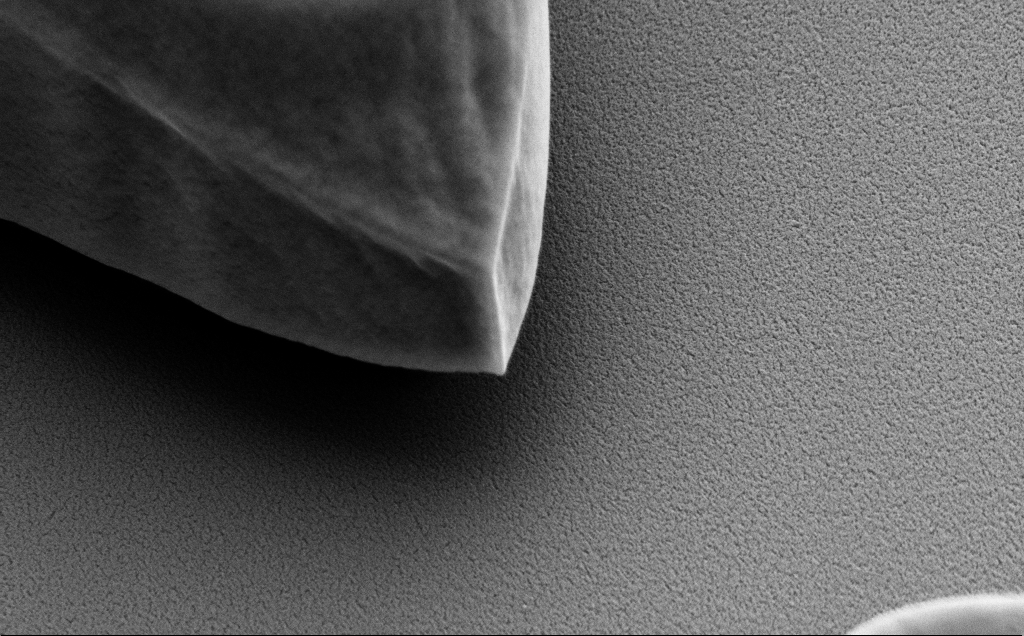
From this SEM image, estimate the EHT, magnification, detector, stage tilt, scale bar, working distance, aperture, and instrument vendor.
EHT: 5 kV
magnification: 27.77 K X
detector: SE2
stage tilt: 40°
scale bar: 2000 nm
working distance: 9 mm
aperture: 30 µm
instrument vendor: Zeiss